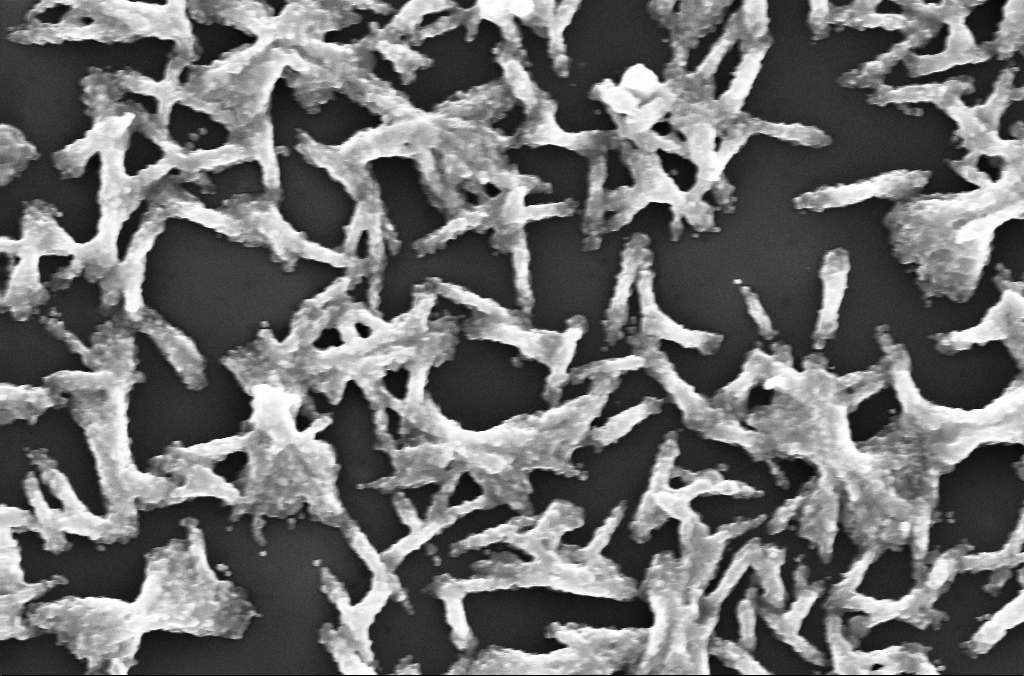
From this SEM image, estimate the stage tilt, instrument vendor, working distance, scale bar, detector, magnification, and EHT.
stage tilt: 20°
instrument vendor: Zeiss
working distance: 2.8 mm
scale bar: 2000 nm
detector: InLens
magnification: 10 K X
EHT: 20 kV